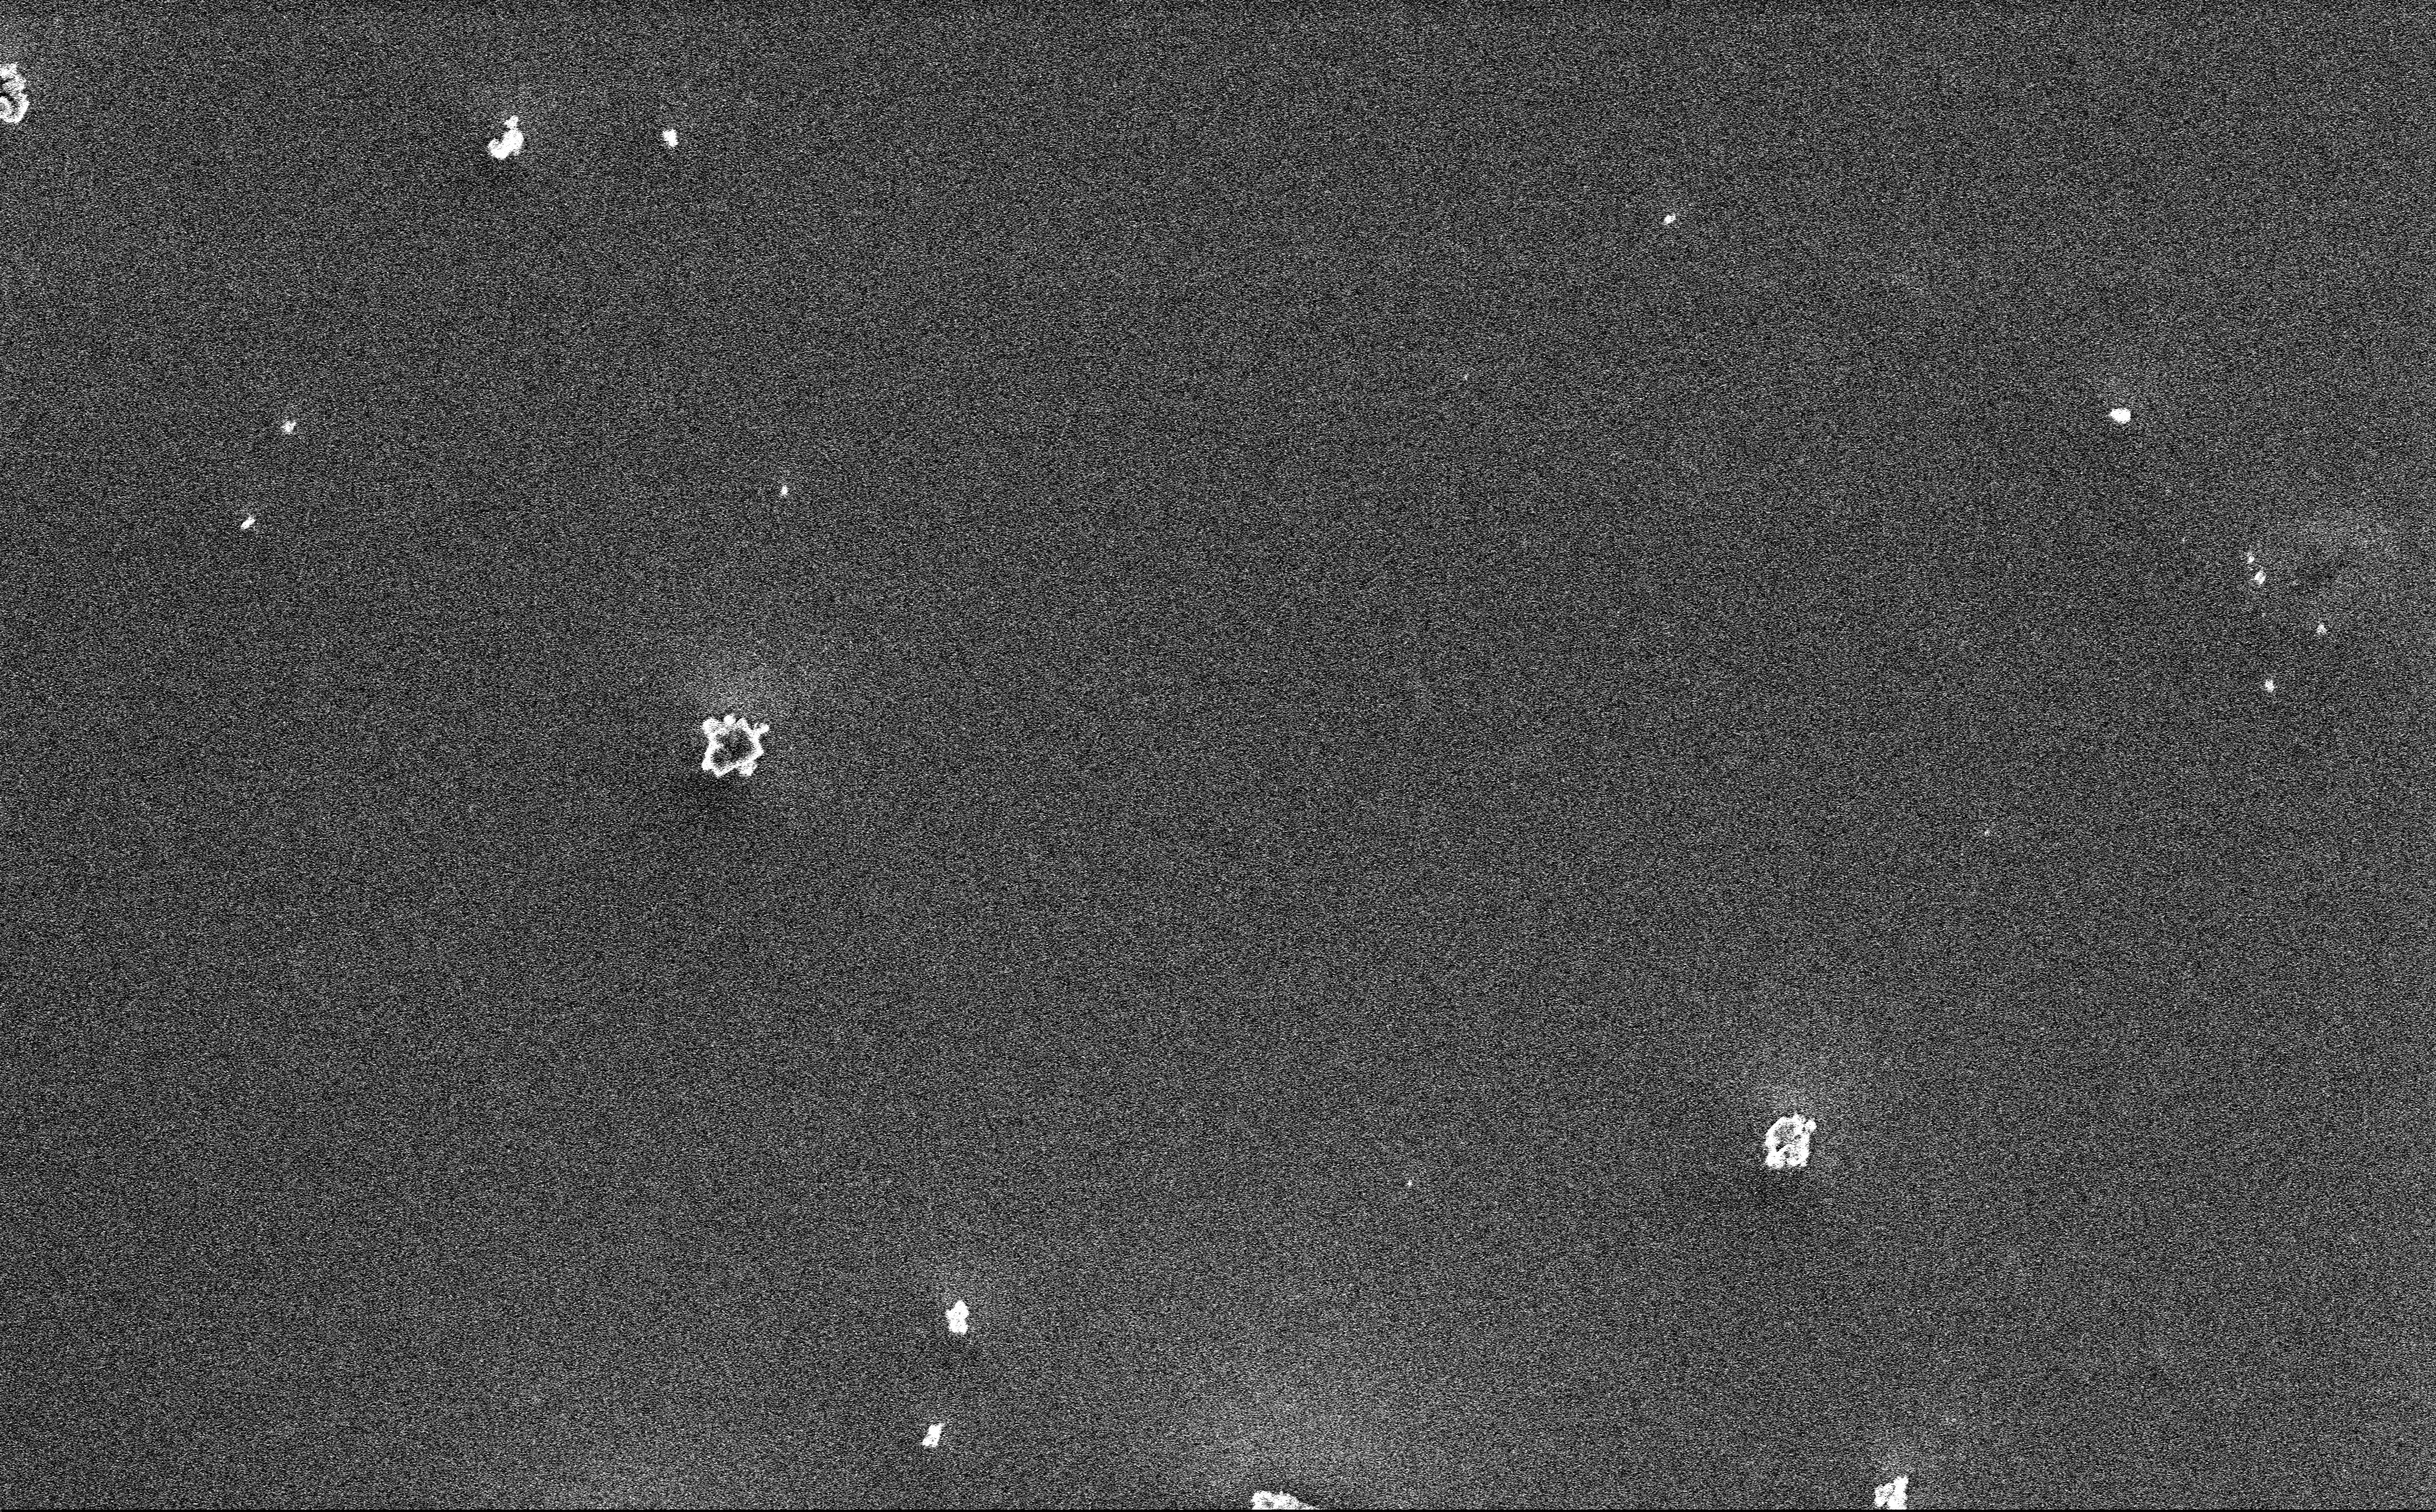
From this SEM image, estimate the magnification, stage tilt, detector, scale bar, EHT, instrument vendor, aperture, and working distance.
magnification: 12.85 K X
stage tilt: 0°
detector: InLens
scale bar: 2000 nm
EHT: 3 kV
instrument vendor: Zeiss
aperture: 30 µm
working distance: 3 mm